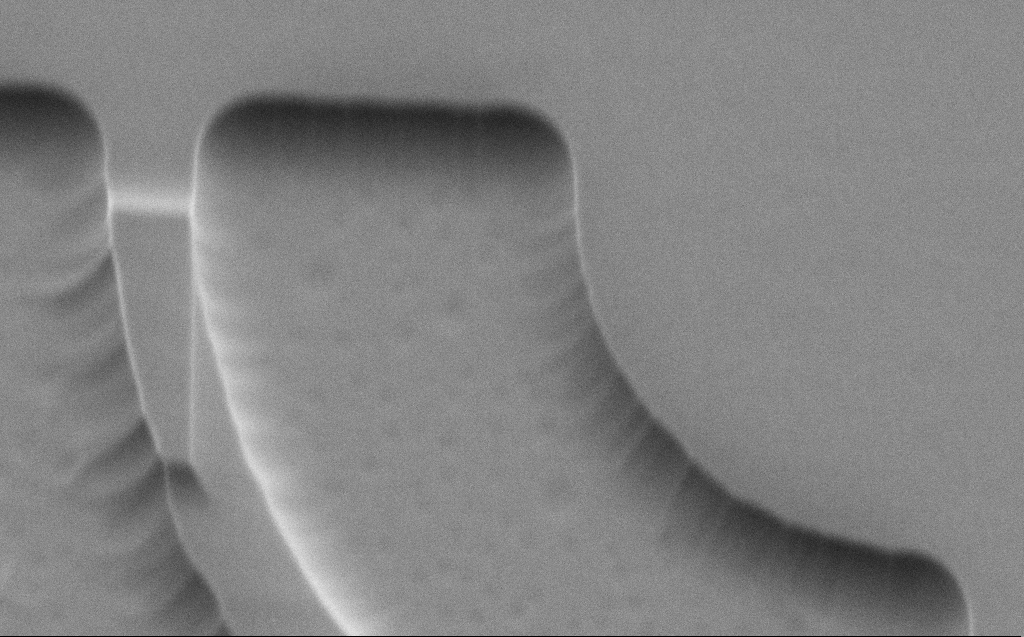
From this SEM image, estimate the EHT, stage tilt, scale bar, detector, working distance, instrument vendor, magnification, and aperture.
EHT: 3 kV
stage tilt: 45°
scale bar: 2000 nm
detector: SE2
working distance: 5 mm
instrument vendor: Zeiss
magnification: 26.7 K X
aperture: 30 µm